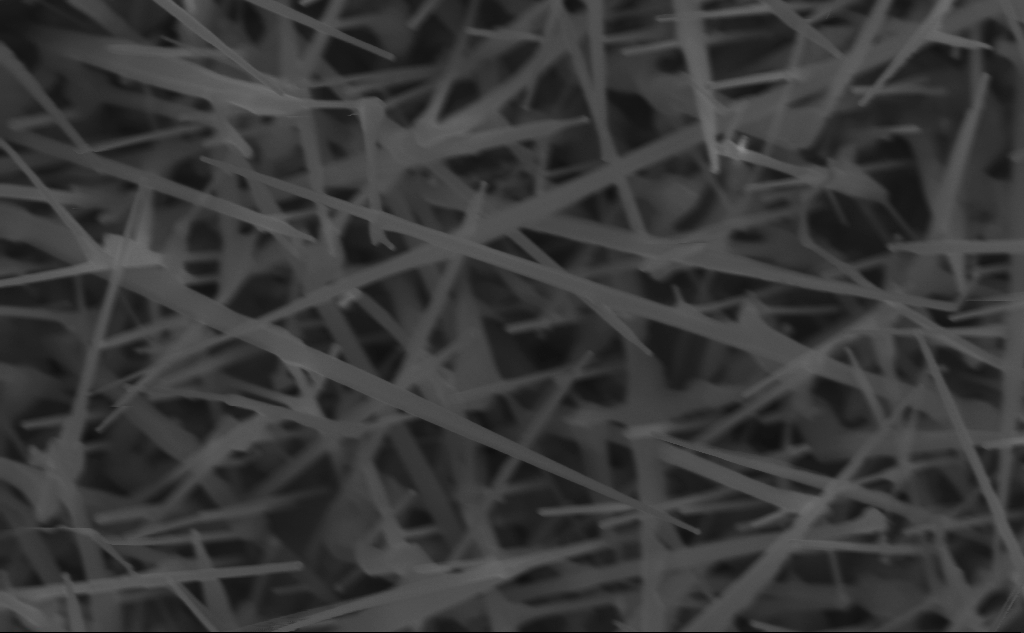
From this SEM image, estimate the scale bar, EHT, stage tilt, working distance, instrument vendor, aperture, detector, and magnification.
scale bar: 200 nm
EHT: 10 kV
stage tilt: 0°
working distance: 7 mm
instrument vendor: Zeiss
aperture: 30 µm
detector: InLens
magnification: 77.65 K X